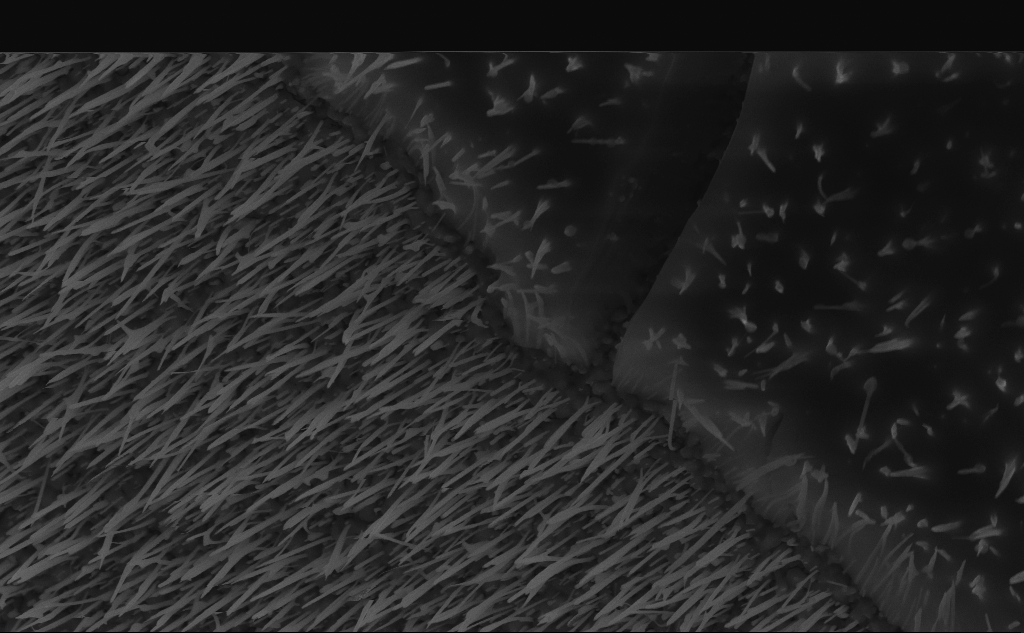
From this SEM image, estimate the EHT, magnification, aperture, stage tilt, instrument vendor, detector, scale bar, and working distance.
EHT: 10 kV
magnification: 30.35 K X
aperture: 30 µm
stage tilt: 45°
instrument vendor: Zeiss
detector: InLens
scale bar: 2000 nm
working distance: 6 mm